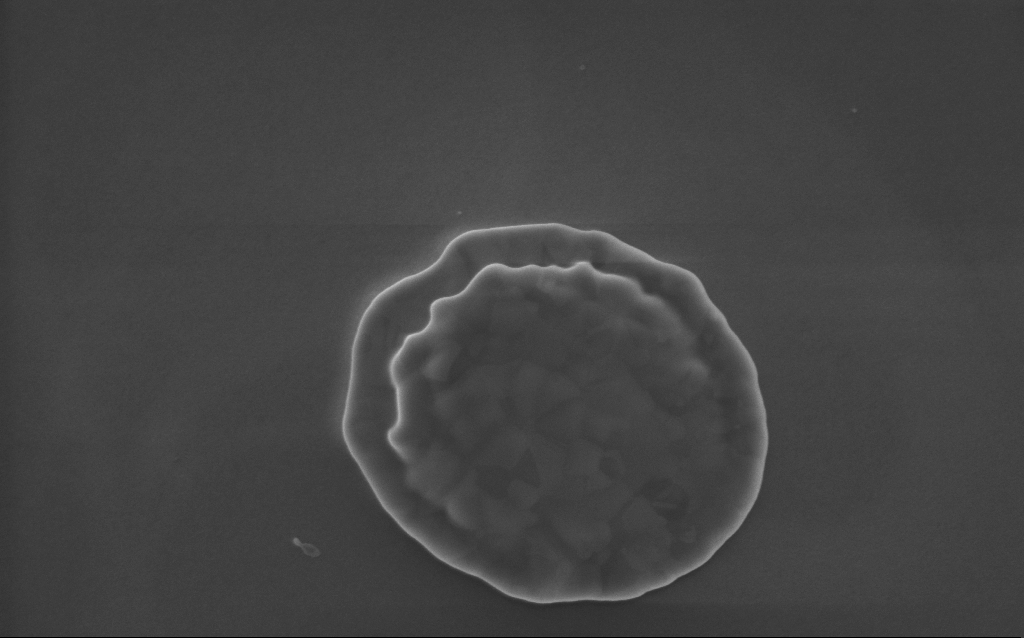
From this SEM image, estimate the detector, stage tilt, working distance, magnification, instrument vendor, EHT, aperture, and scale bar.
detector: InLens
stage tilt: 0°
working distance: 3 mm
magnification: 82 K X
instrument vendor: Zeiss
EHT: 5 kV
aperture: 30 µm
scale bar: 200 nm